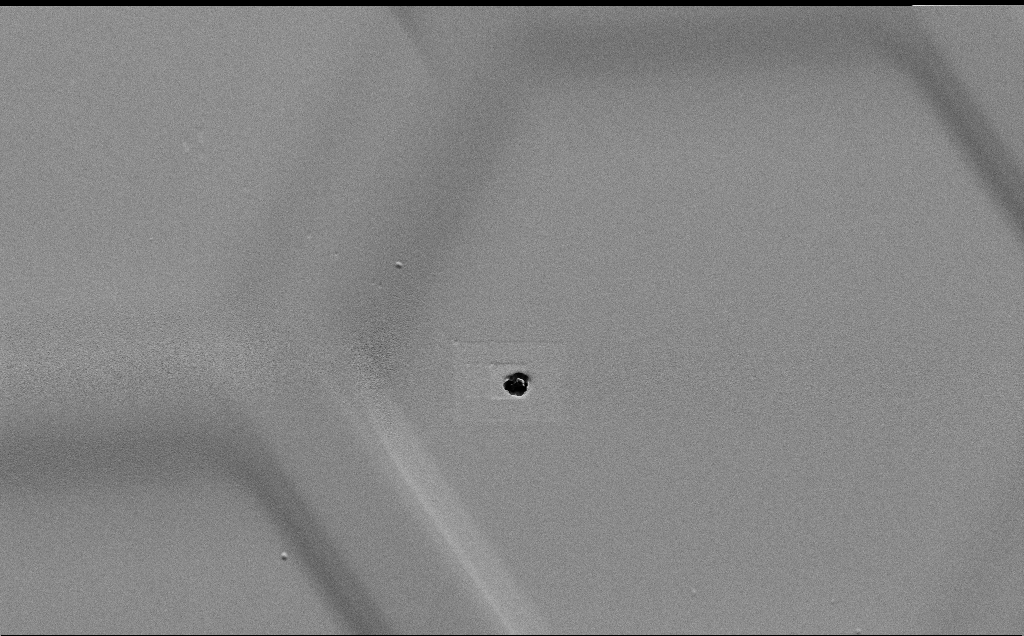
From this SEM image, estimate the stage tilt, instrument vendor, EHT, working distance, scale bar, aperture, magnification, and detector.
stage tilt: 45°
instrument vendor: Zeiss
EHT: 1.5 kV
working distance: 4 mm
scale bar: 10000 nm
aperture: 30 µm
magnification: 4.89 K X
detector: SE2